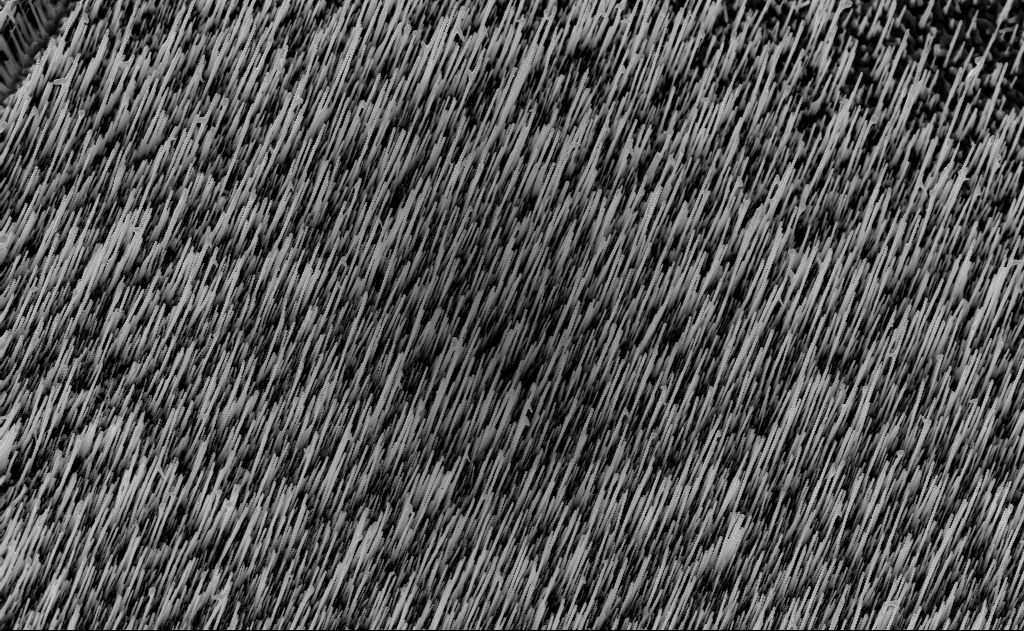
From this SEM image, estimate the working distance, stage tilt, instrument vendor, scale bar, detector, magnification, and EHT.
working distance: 7 mm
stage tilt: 0°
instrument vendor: Zeiss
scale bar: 2000 nm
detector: InLens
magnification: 10 K X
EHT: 10 kV